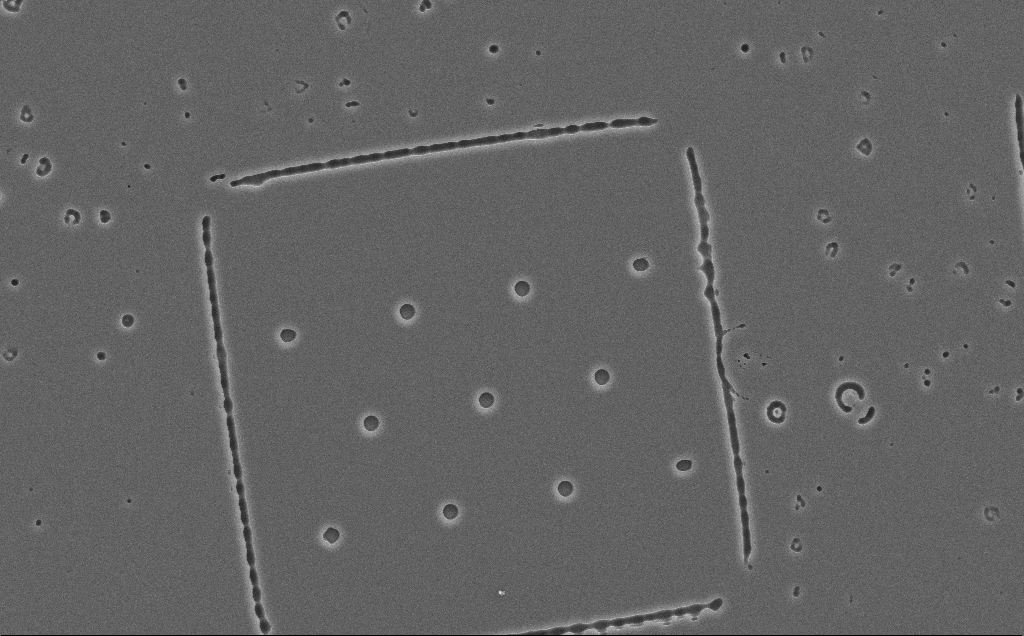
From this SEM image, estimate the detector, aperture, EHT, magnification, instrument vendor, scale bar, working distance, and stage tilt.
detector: SE2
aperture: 30 µm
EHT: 10 kV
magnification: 2.13 K X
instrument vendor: Zeiss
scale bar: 20000 nm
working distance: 12 mm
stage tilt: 0°